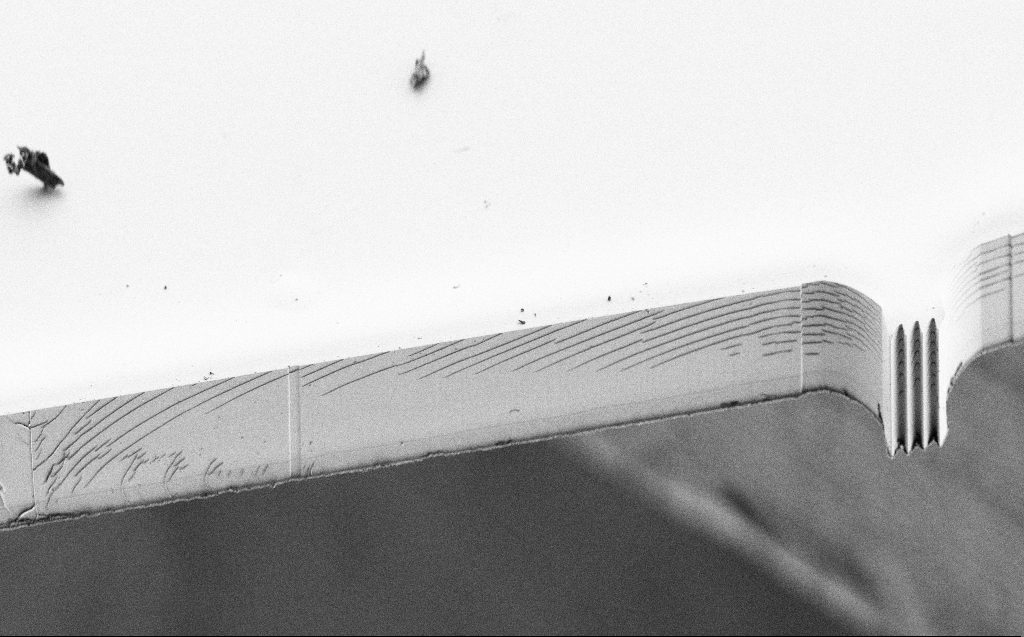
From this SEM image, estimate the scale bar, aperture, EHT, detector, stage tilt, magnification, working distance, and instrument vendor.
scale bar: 100000 nm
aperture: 30 µm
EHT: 3 kV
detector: SE2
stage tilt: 45°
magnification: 0.534 K X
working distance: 4 mm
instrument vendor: Zeiss